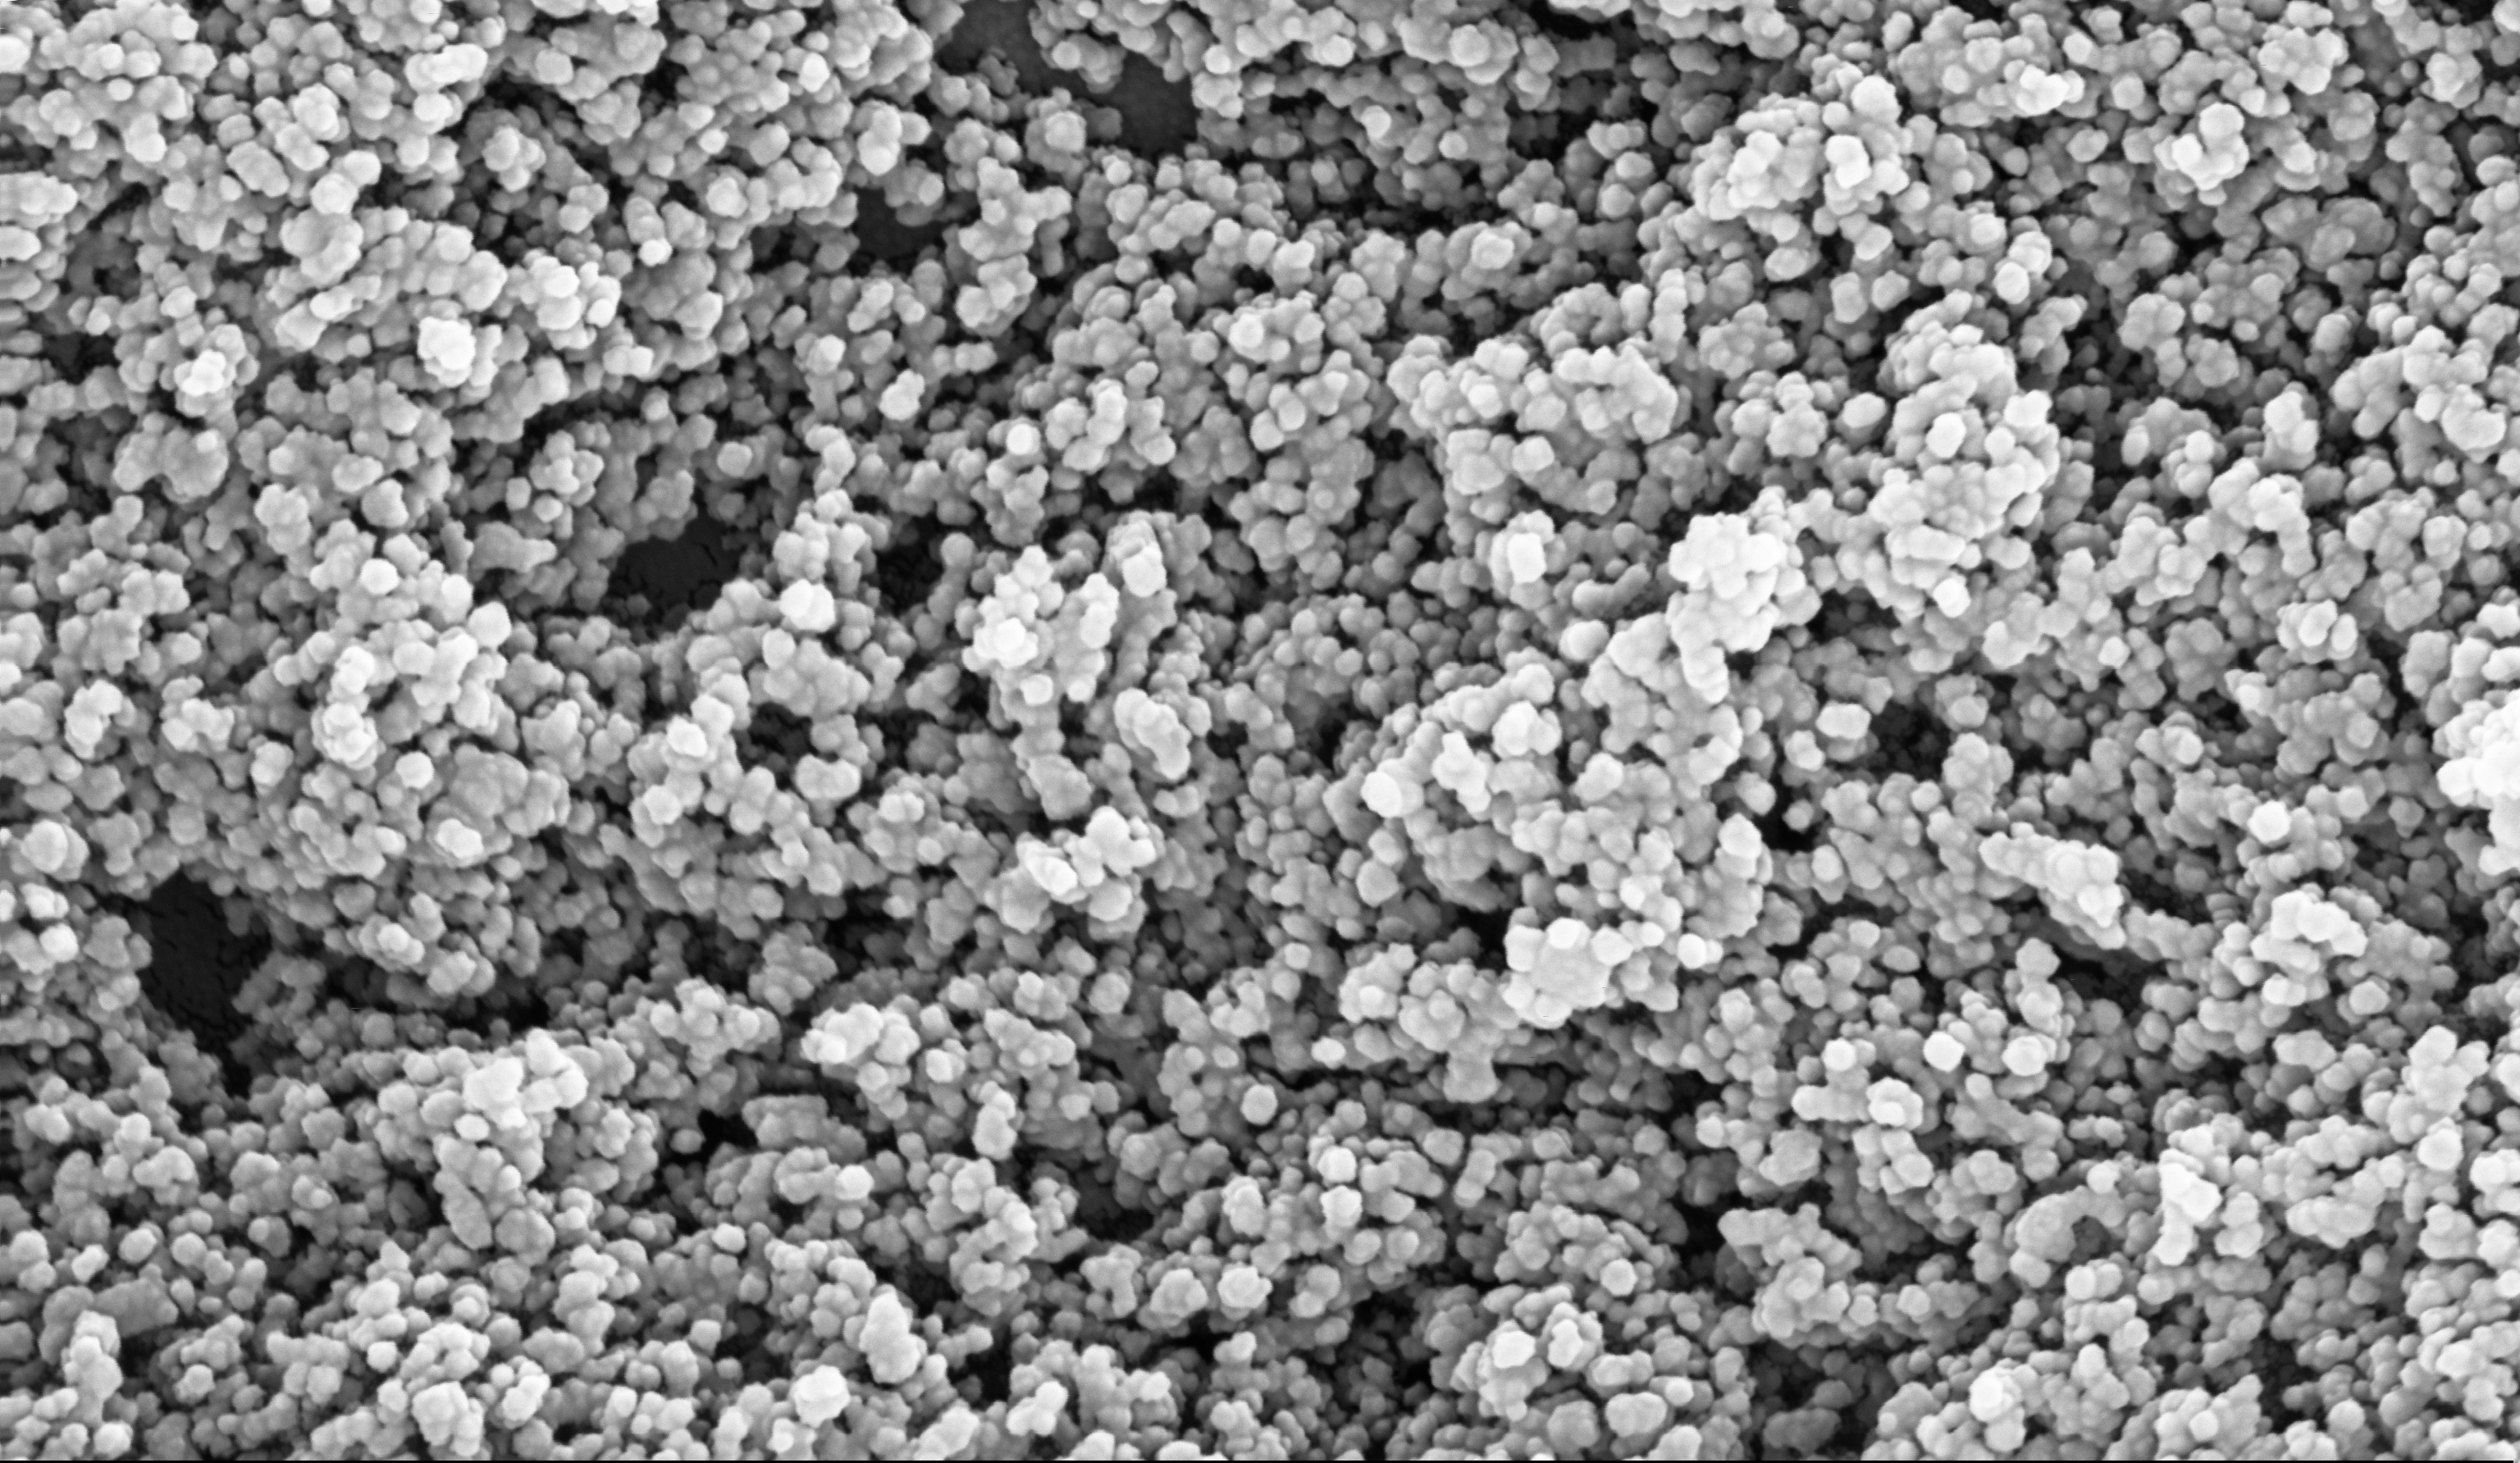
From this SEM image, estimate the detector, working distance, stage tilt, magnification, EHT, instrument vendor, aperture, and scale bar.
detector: InLens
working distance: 5.1 mm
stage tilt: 0°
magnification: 135 K X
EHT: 10 kV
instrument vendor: Zeiss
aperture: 30 µm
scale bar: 200 nm